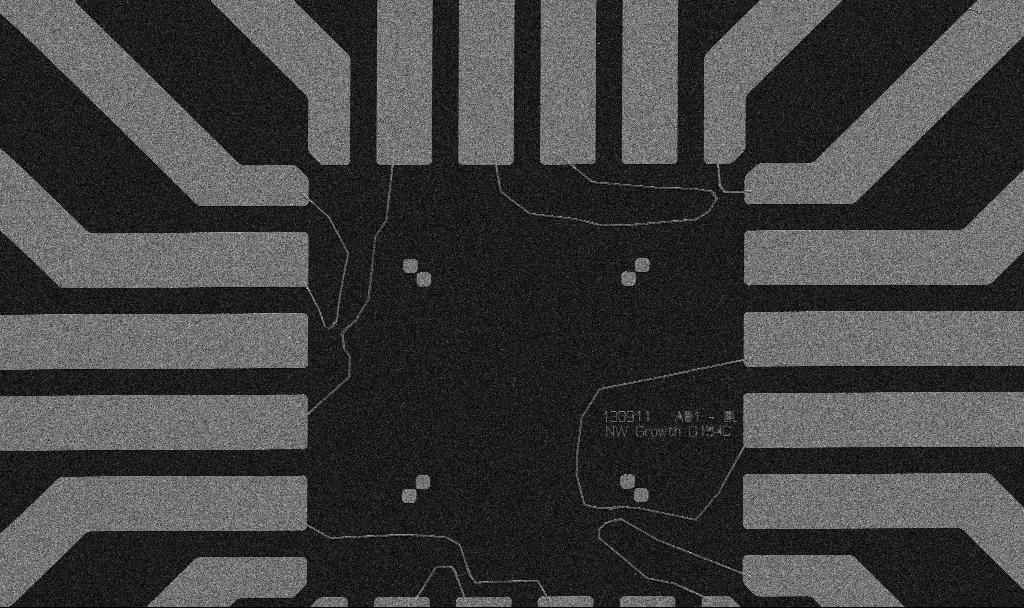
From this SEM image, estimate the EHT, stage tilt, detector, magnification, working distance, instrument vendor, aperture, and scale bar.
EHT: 5 kV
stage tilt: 0°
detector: SE2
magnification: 1 K X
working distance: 10.7 mm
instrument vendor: Zeiss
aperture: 30 µm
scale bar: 20000 nm